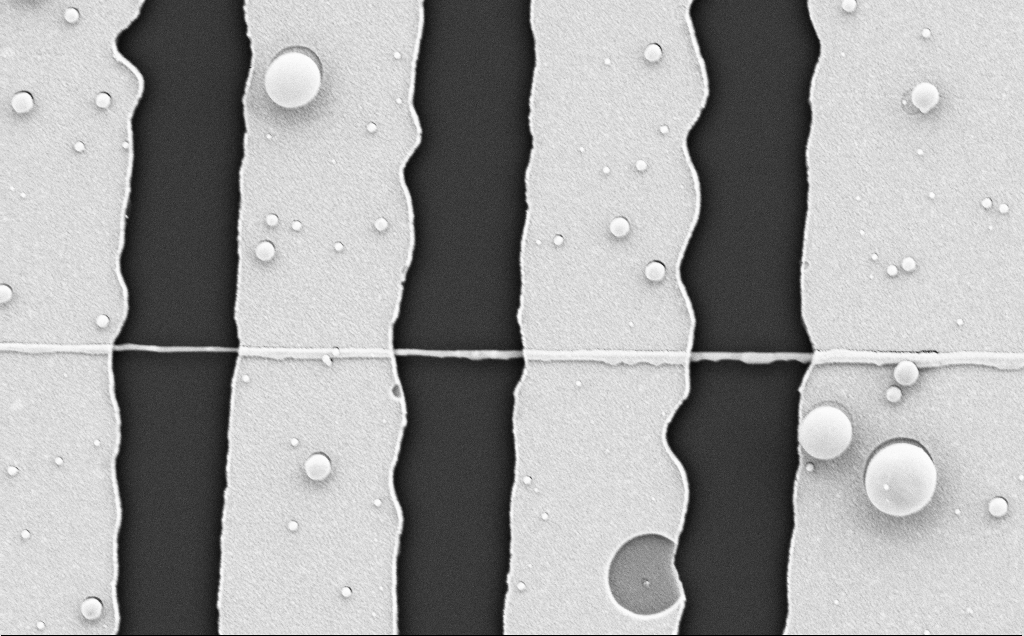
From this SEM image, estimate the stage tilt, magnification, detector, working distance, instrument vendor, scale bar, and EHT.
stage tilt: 0°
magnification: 25.98 K X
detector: SE2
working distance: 10 mm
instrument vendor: Zeiss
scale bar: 2000 nm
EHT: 5 kV